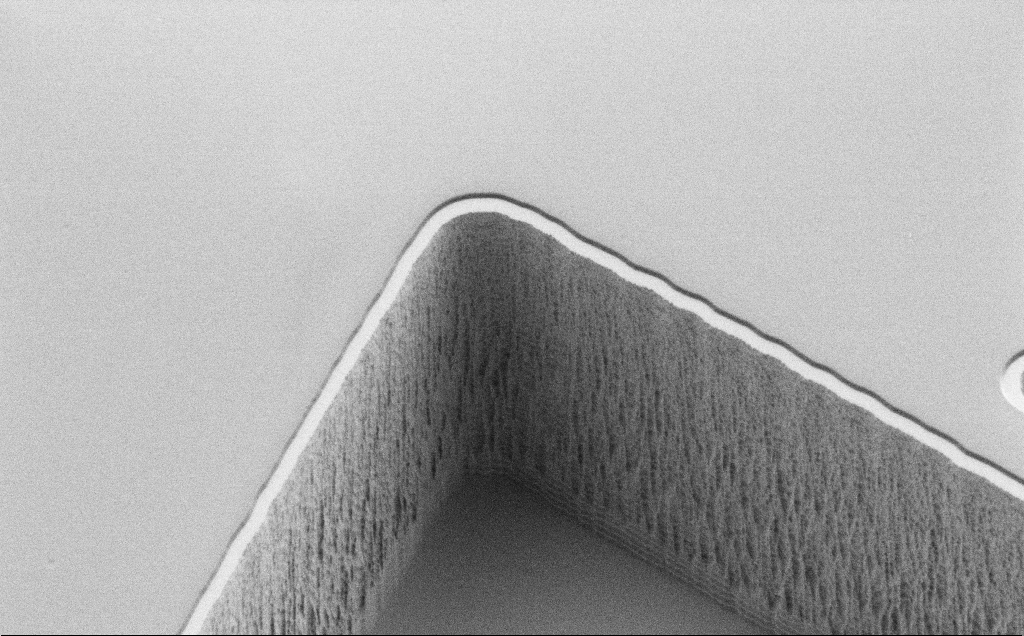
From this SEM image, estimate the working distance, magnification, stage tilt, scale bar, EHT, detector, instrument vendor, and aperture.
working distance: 7 mm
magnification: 6.82 K X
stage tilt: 45°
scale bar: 10000 nm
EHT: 1.2 kV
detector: SE2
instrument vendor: Zeiss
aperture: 30 µm